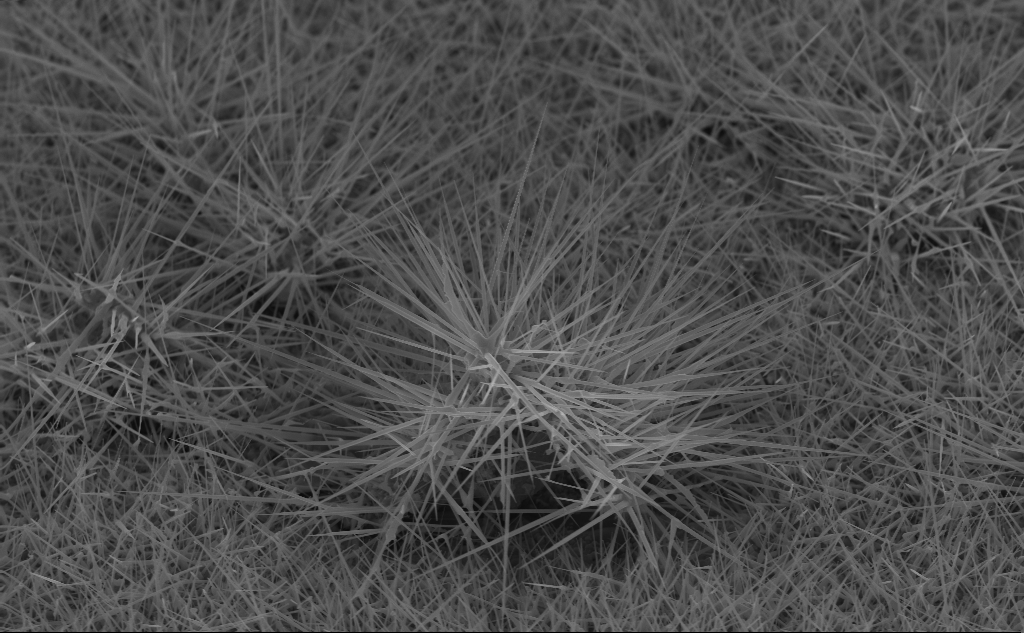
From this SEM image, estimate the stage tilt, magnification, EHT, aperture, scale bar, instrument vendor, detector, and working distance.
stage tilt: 45°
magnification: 12.07 K X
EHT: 10 kV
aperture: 30 µm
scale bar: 2000 nm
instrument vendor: Zeiss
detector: InLens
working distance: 5 mm